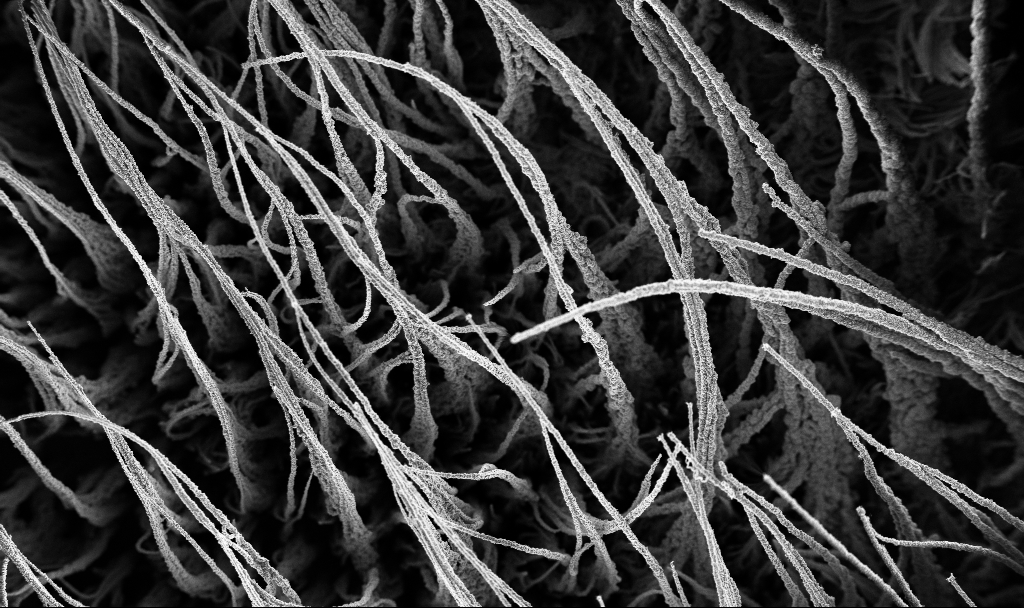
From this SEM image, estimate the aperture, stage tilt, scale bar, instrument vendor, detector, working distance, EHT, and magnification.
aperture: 30 µm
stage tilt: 0°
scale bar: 100000 nm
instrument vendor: Zeiss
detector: InLens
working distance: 2.9 mm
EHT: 3 kV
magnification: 0.15 K X